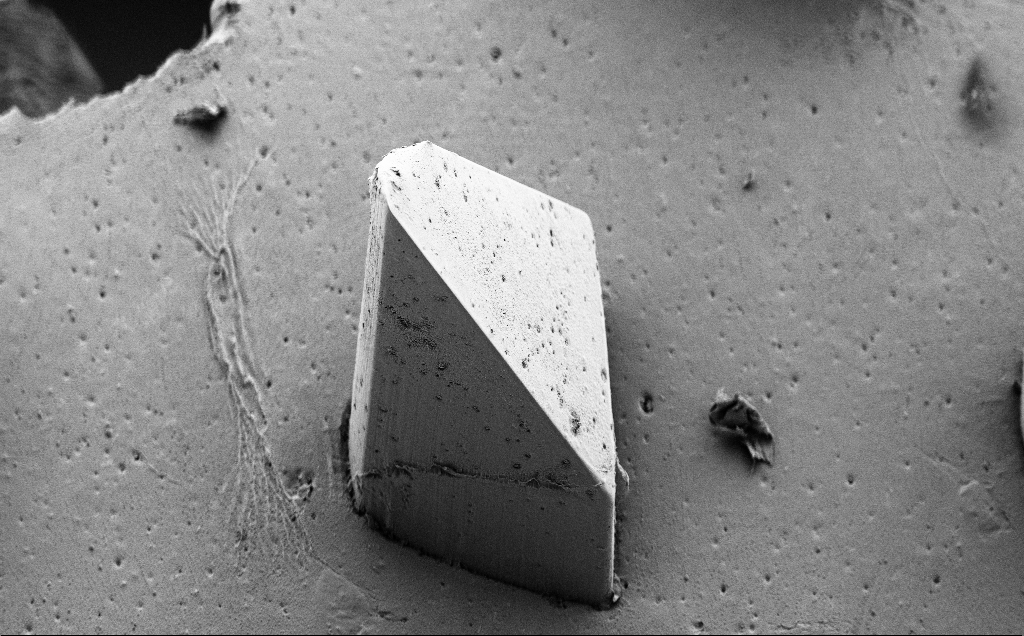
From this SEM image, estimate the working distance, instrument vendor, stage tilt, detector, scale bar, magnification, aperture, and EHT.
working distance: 8 mm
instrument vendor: Zeiss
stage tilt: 40°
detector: SE2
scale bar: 100000 nm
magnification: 0.27 K X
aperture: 30 µm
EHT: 5 kV